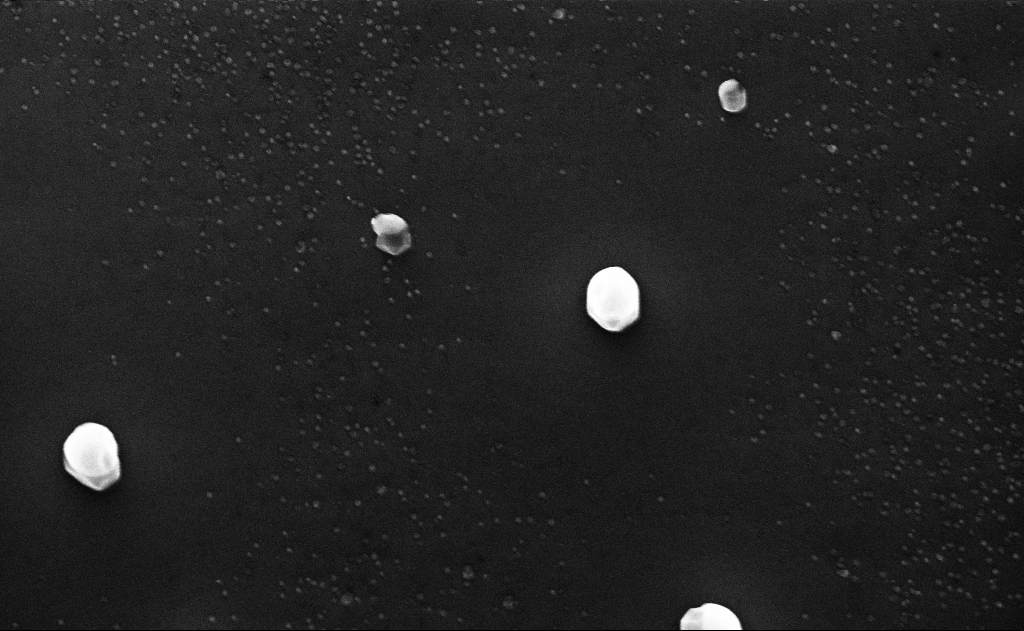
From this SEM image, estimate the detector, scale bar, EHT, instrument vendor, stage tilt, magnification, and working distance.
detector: InLens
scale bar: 1000 nm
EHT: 10 kV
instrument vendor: Zeiss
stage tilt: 0°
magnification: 50 K X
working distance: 10 mm